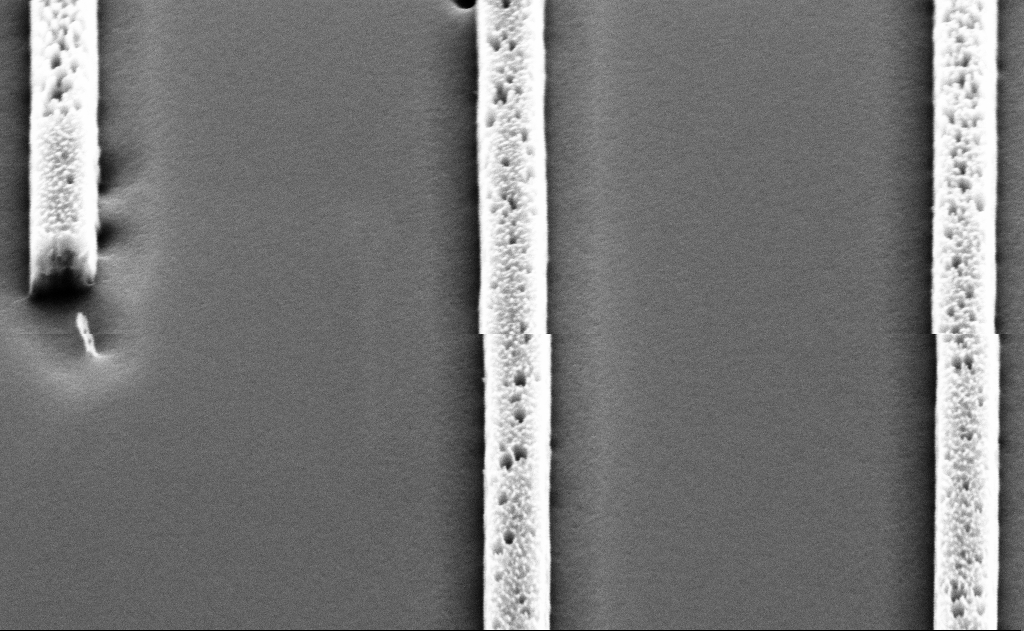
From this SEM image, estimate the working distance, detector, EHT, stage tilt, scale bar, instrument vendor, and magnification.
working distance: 11 mm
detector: SE2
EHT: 5 kV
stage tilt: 45°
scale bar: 1000 nm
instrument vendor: Zeiss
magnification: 41.19 K X